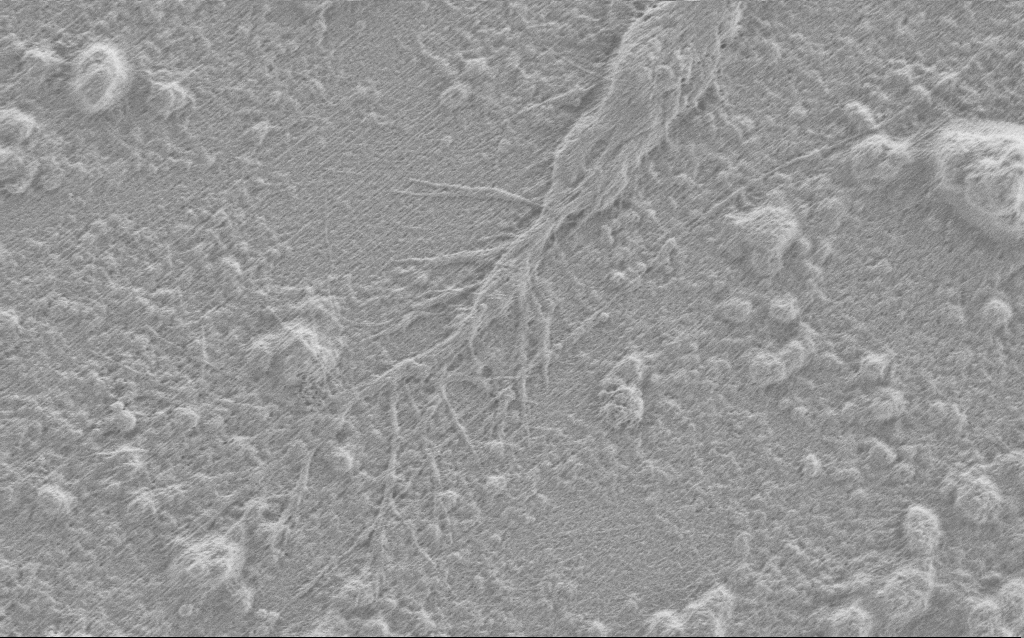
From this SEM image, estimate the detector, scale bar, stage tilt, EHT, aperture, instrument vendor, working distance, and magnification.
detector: SE2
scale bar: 2000 nm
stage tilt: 0°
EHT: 0.9 kV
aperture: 30 µm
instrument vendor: Zeiss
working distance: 4 mm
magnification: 7.5 K X